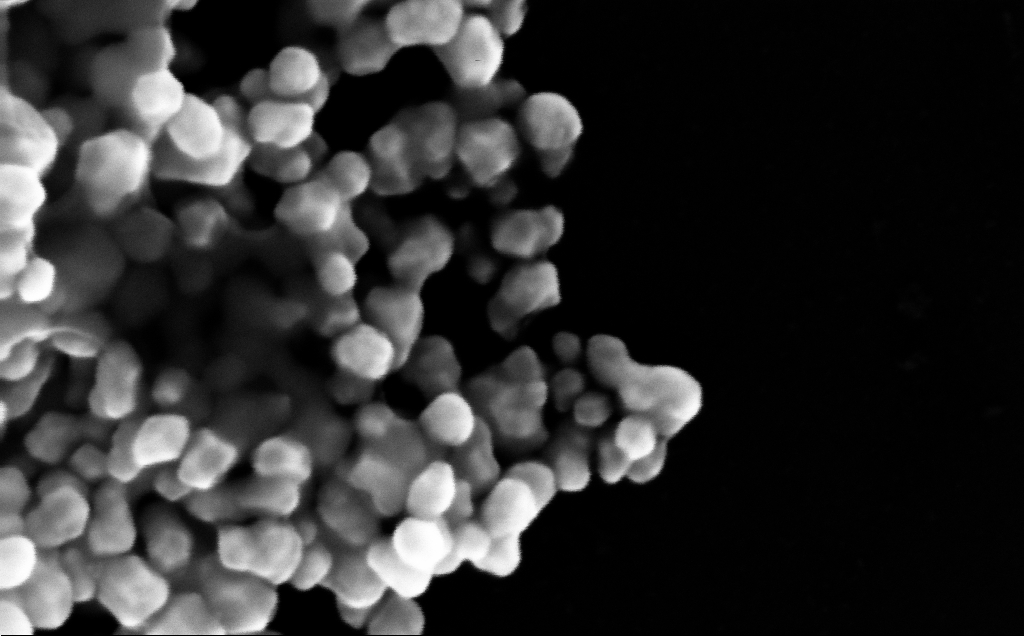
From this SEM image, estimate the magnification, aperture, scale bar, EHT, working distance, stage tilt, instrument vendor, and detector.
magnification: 510.11 K X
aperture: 30 µm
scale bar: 100 nm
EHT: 10 kV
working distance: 4 mm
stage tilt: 0°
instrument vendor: Zeiss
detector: InLens